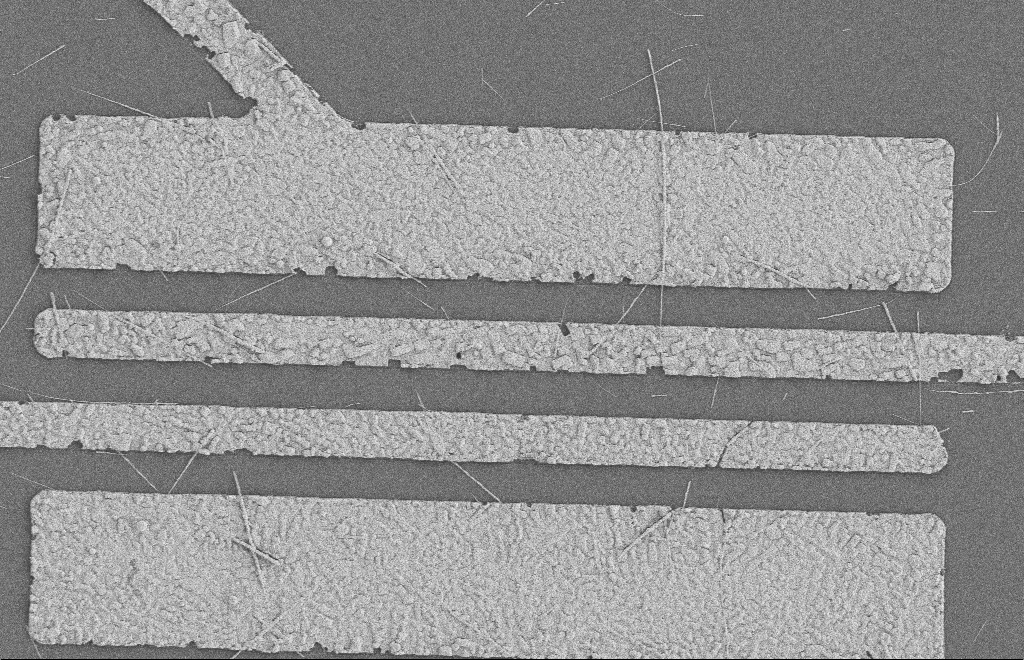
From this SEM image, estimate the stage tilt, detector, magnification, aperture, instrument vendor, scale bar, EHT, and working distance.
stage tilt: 0°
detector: SE2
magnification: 5.54 K X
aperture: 20 µm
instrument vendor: Zeiss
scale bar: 2000 nm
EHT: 2 kV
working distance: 8 mm